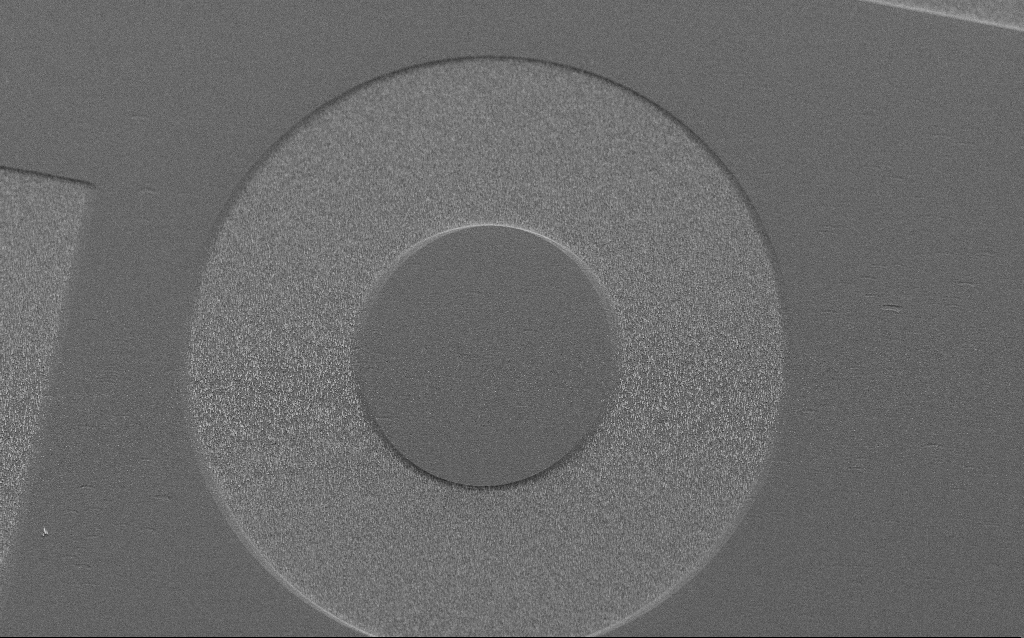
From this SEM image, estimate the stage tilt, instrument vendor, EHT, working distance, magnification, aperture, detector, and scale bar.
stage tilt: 45°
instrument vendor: Zeiss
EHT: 5 kV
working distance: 8 mm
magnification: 0.611 K X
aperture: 30 µm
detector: SE2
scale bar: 100000 nm